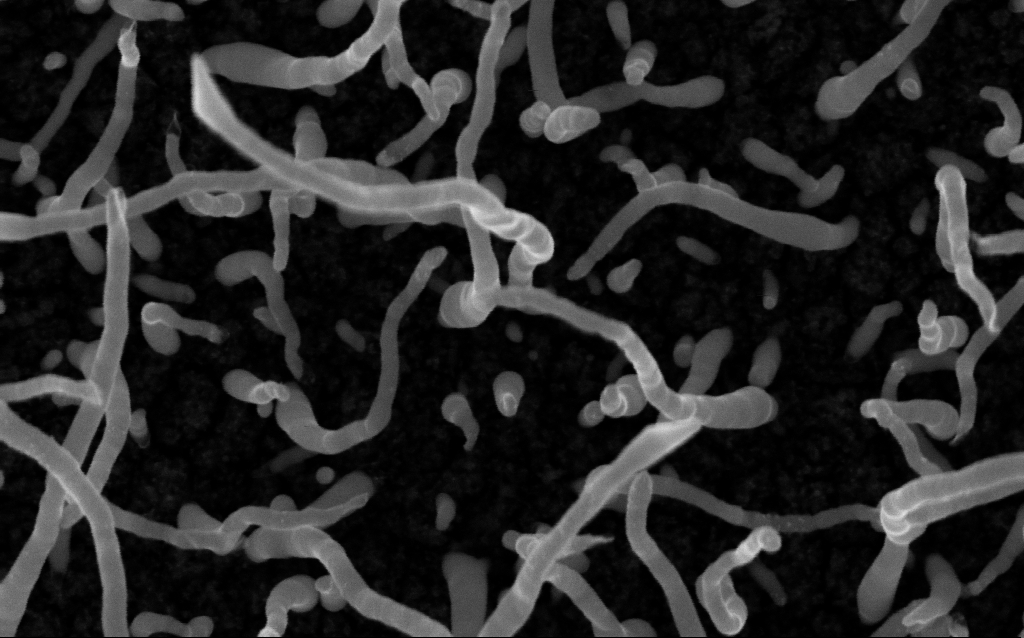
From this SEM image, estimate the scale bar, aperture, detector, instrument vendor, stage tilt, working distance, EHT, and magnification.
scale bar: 100 nm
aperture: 30 µm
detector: InLens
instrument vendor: Zeiss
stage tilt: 0°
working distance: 2 mm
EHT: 5 kV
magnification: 200 K X